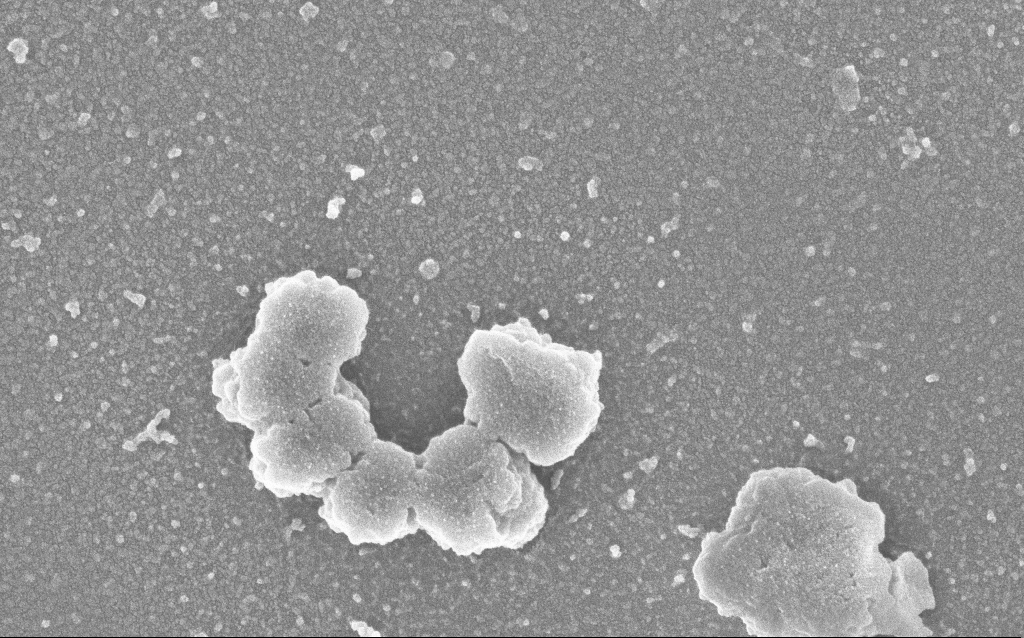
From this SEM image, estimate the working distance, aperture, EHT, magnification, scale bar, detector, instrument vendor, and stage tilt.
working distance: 2.5 mm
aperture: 30 µm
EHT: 3 kV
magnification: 100 K X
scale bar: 200 nm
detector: InLens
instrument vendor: Zeiss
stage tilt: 0°